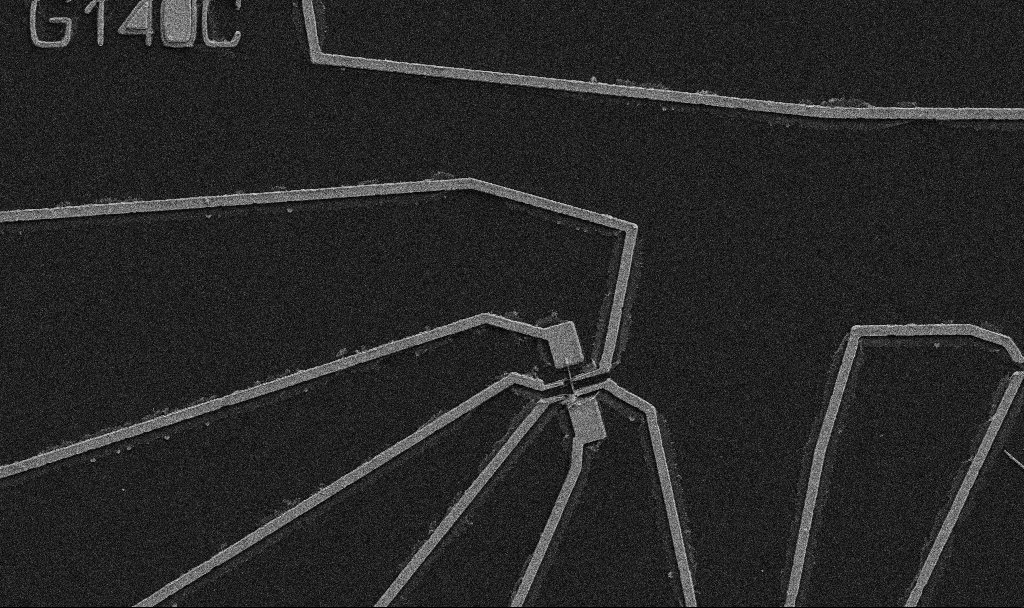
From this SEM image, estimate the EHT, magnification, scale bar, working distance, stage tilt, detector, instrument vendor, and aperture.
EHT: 5 kV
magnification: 5 K X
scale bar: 10000 nm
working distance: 10.7 mm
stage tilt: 0°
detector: SE2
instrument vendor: Zeiss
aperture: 30 µm